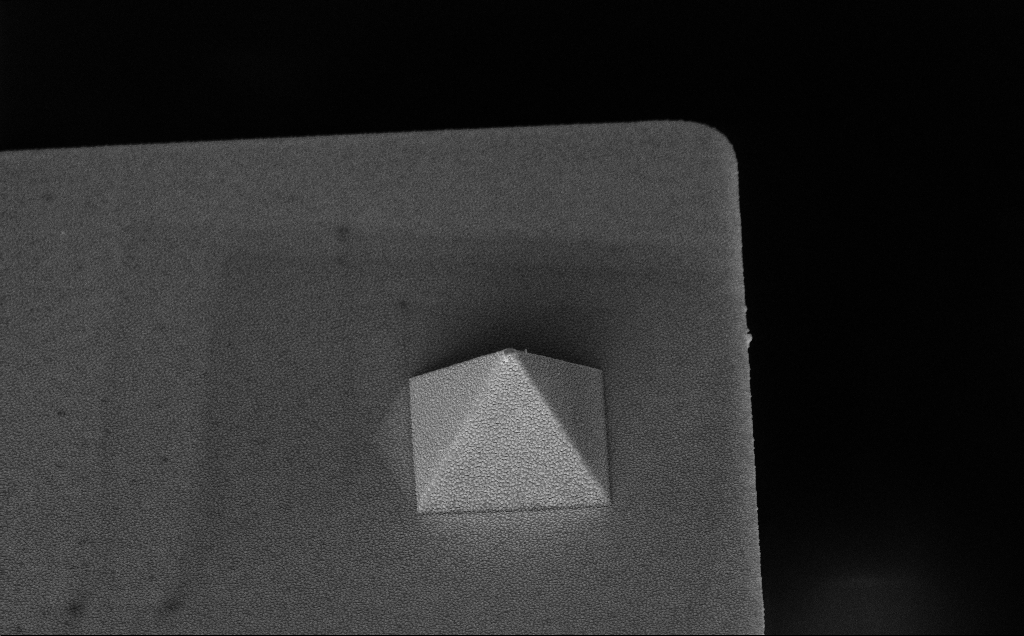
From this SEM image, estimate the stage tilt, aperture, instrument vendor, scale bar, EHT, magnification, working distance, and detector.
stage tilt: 45°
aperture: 30 µm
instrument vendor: Zeiss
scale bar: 1000 nm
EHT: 10 kV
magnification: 13.82 K X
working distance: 11 mm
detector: InLens